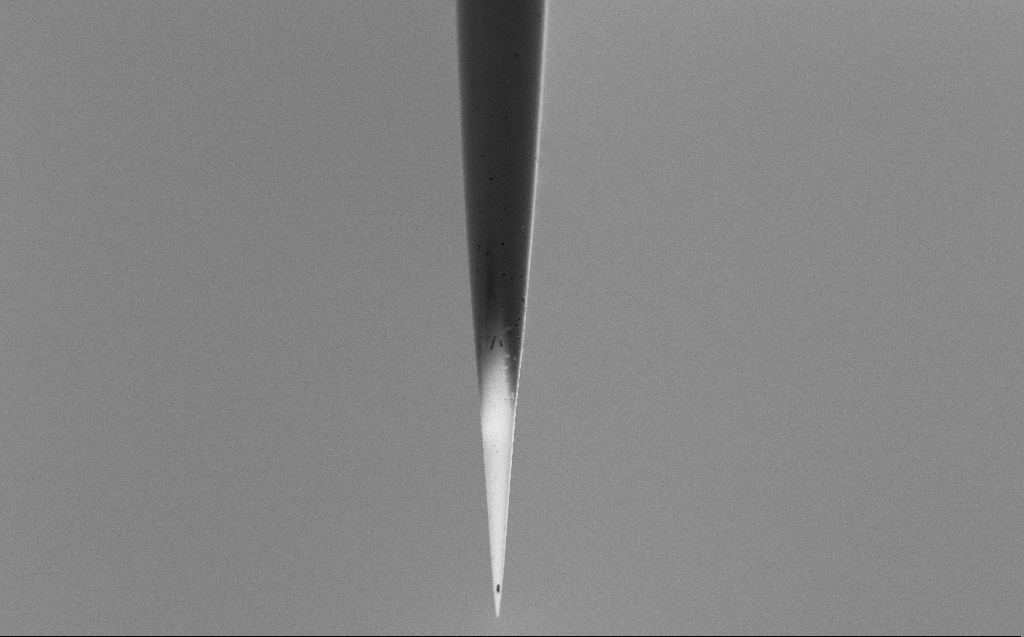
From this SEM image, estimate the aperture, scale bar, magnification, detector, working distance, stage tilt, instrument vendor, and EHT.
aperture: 30 µm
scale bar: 10000 nm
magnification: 1.91 K X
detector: SE2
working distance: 4 mm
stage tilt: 45°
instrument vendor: Zeiss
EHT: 5 kV